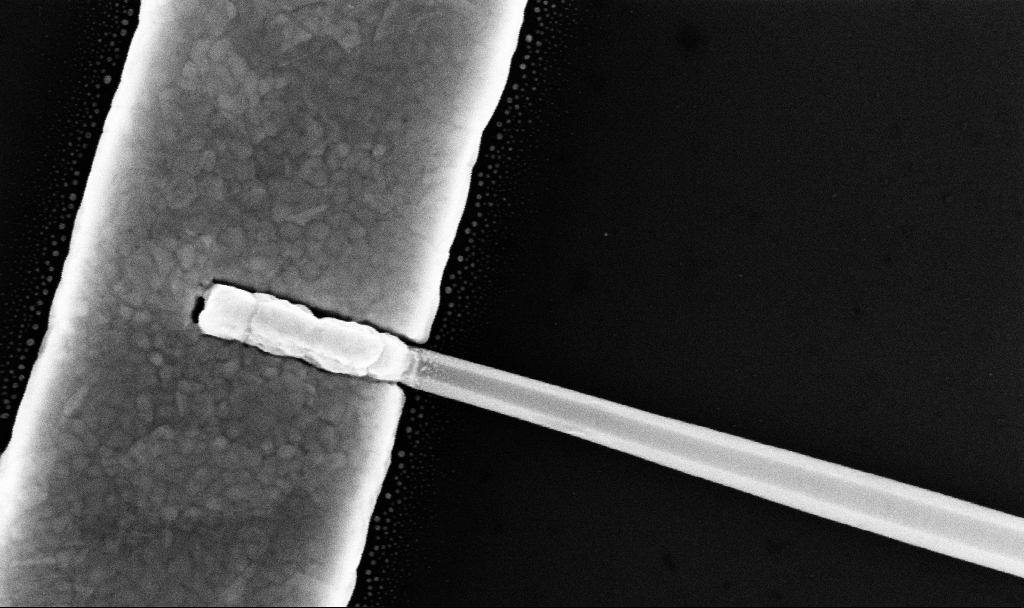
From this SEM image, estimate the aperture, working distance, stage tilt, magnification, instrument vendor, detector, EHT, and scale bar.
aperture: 30 µm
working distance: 7.7 mm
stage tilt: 0°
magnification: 158.26 K X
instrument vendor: Zeiss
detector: InLens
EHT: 10 kV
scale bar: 200 nm